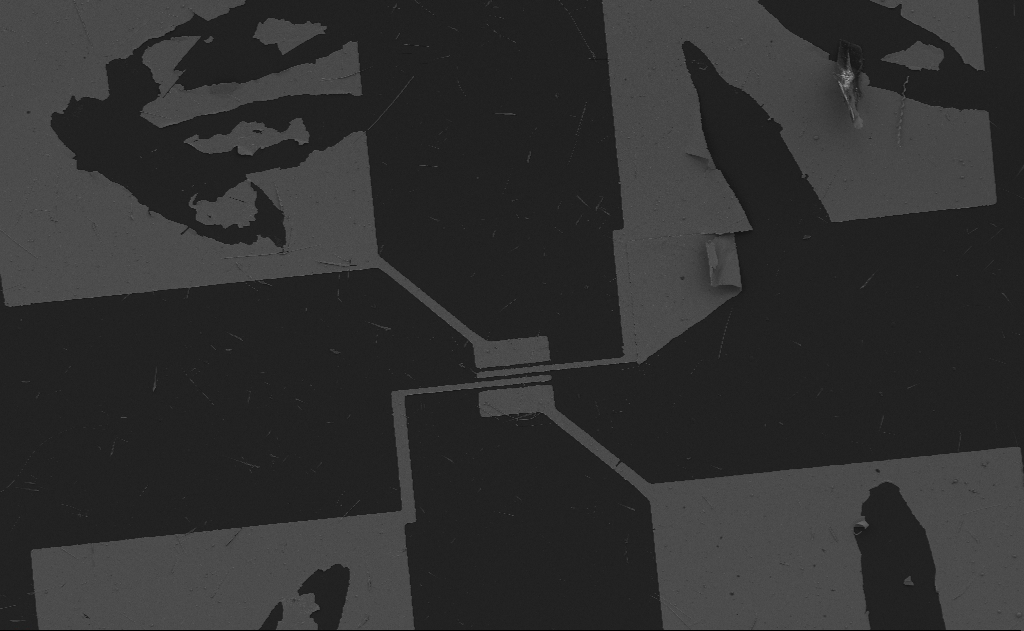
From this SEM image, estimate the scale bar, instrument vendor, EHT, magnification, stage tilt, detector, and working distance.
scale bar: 20000 nm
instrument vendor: Zeiss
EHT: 5 kV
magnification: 0.907 K X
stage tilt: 0°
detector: SE2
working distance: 13 mm